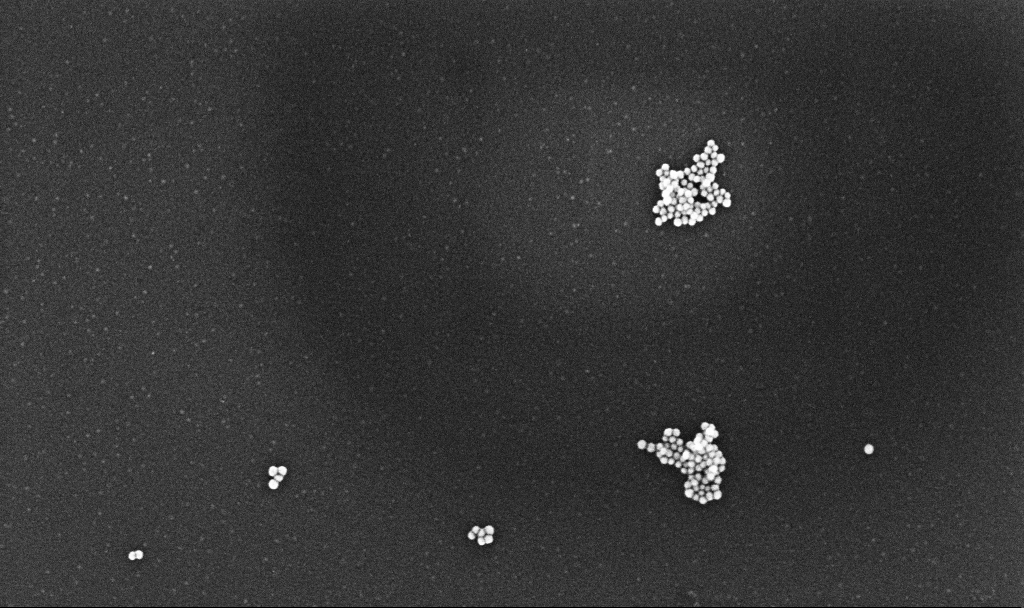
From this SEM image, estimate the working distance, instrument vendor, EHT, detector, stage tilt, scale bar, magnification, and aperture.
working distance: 3.1 mm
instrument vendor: Zeiss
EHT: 10 kV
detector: InLens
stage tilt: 0°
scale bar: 200 nm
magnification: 122.92 K X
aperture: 30 µm